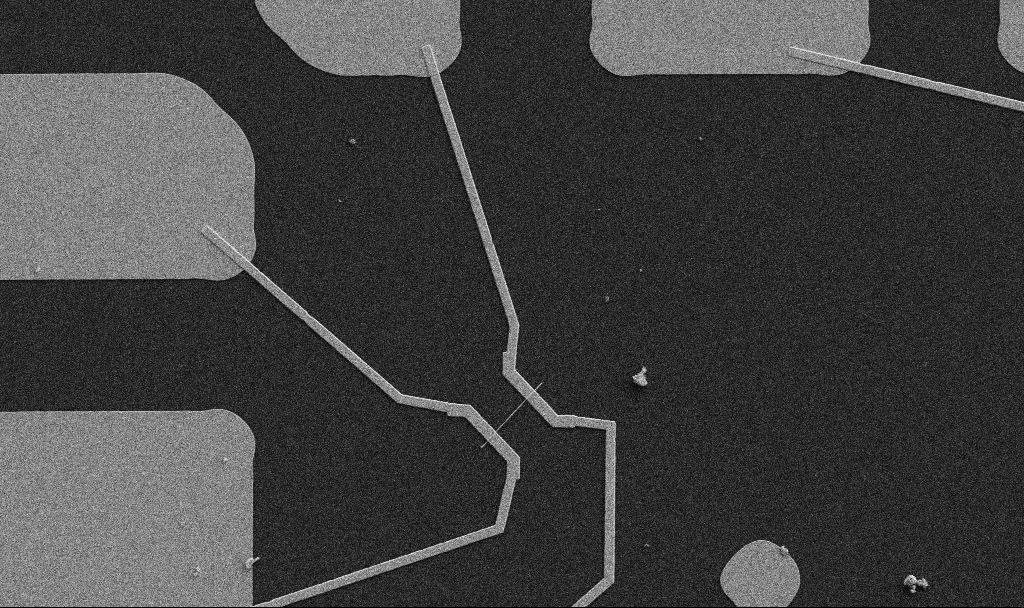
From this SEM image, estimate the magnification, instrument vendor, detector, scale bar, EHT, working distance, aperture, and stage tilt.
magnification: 5 K X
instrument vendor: Zeiss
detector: SE2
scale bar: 10000 nm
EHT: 5 kV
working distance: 10.7 mm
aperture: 30 µm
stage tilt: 0°